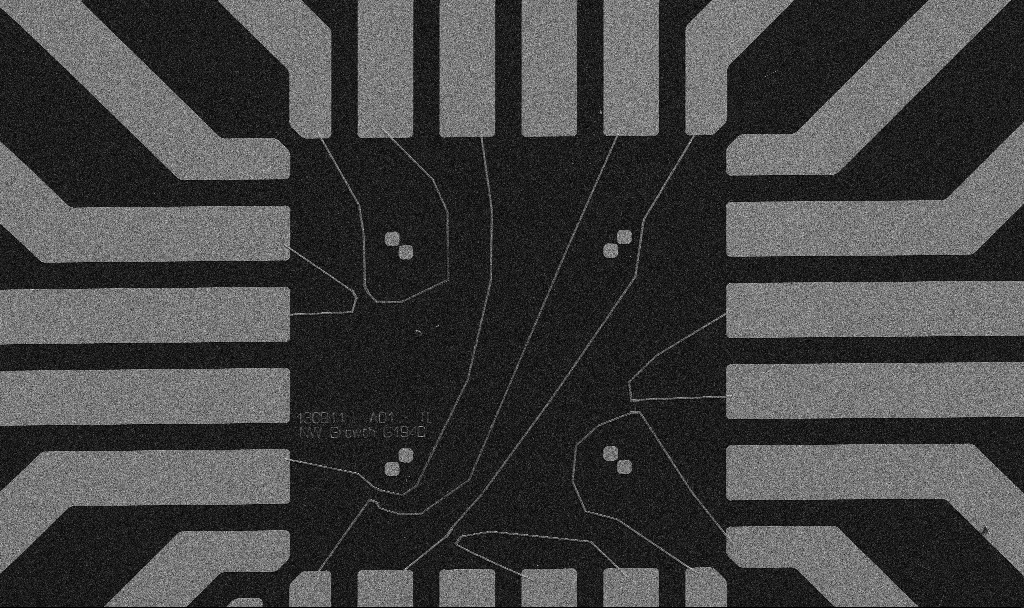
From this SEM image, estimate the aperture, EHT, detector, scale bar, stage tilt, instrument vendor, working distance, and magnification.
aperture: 30 µm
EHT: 5 kV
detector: SE2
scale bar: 20000 nm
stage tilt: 0°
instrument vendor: Zeiss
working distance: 10.7 mm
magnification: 1 K X